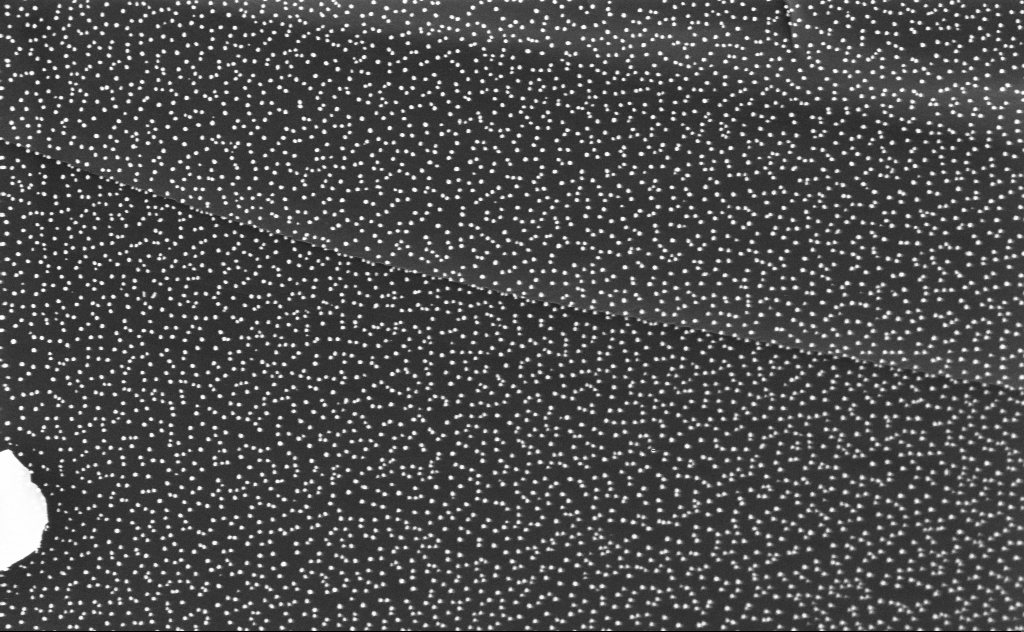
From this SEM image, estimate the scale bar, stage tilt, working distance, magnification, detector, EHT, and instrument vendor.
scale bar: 200 nm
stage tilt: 0°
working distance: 5 mm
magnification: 80 K X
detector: InLens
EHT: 3 kV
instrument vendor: Zeiss